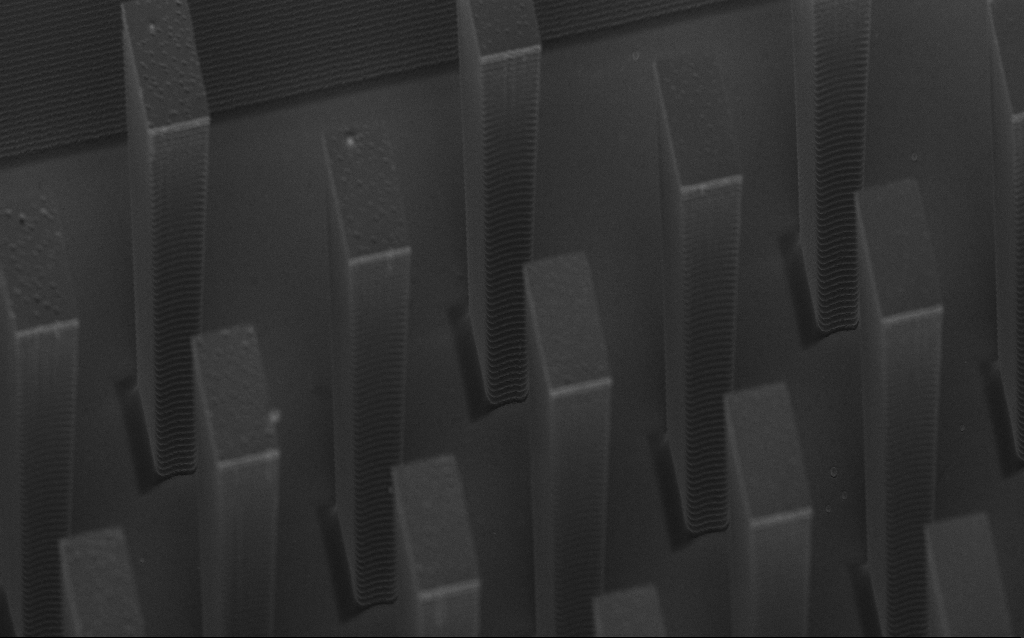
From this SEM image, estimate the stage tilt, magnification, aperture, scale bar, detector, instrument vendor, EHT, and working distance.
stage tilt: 45°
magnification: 6.19 K X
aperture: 30 µm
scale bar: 10000 nm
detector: InLens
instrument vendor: Zeiss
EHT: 5 kV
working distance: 8 mm